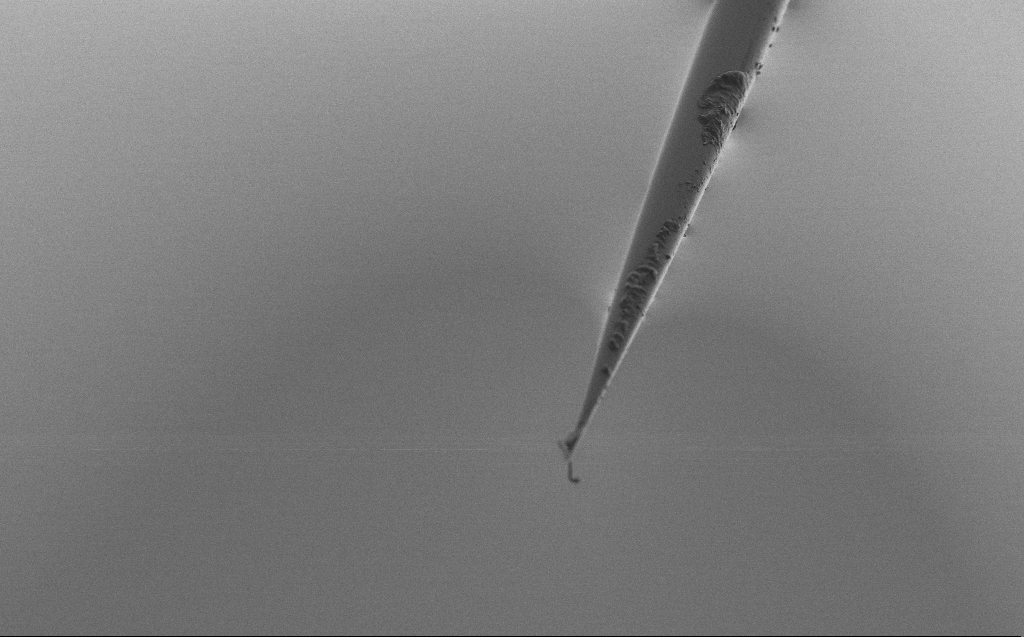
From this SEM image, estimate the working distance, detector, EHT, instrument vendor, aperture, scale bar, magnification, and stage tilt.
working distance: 3 mm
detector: SE2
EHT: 2 kV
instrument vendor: Zeiss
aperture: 30 µm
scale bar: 20000 nm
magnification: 1 K X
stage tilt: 45°